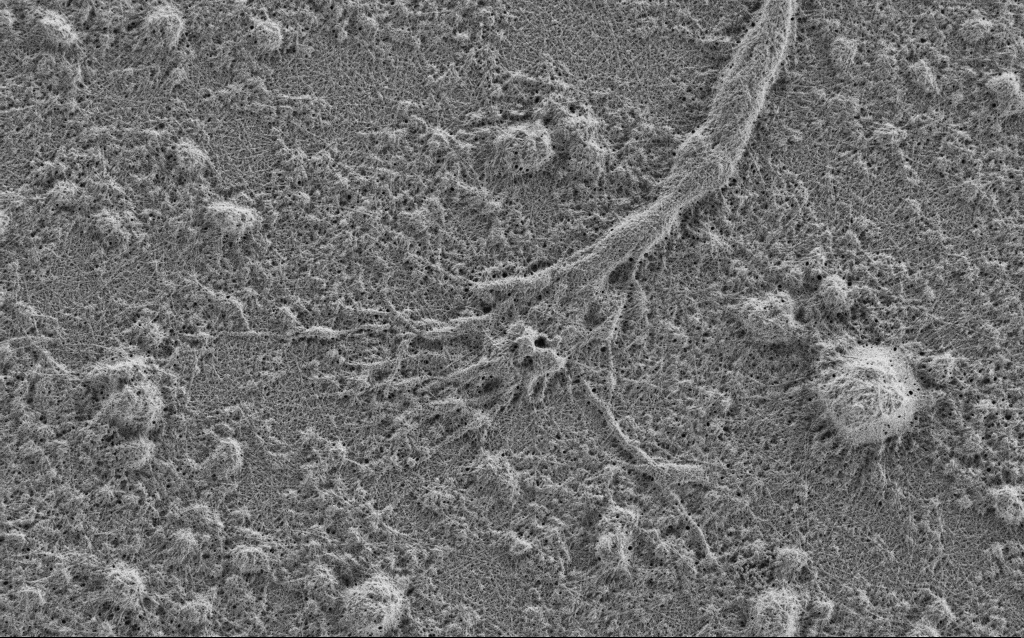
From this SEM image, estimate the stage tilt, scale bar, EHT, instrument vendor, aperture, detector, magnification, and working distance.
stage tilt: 0°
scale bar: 2000 nm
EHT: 0.9 kV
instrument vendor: Zeiss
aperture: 30 µm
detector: SE2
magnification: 10 K X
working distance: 4 mm